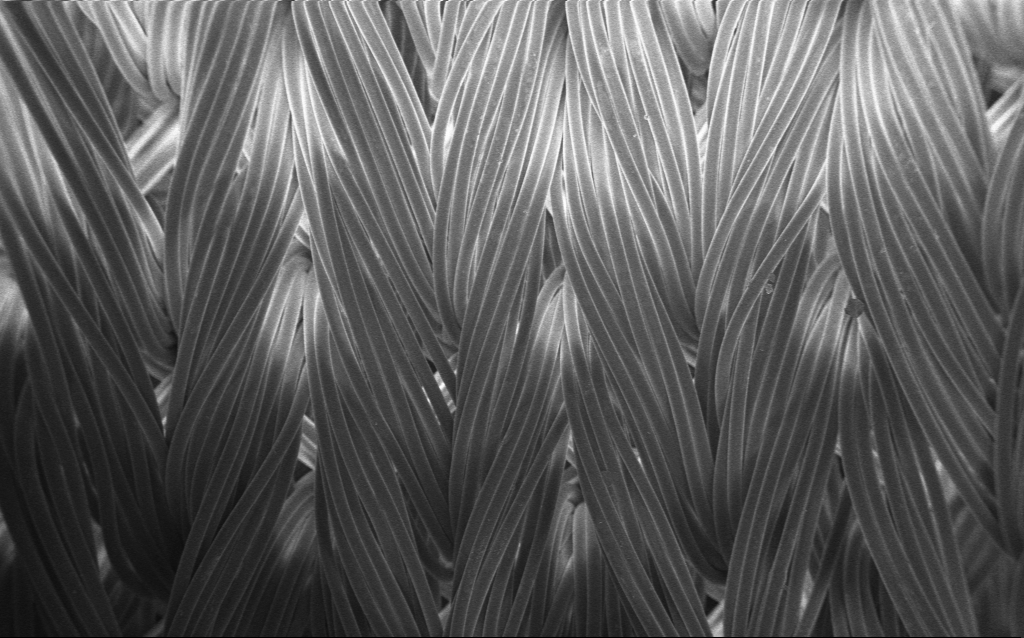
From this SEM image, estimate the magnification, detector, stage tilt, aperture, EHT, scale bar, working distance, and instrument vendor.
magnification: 0.216 K X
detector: InLens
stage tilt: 0°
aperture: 30 µm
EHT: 1 kV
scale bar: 200000 nm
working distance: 4 mm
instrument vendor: Zeiss